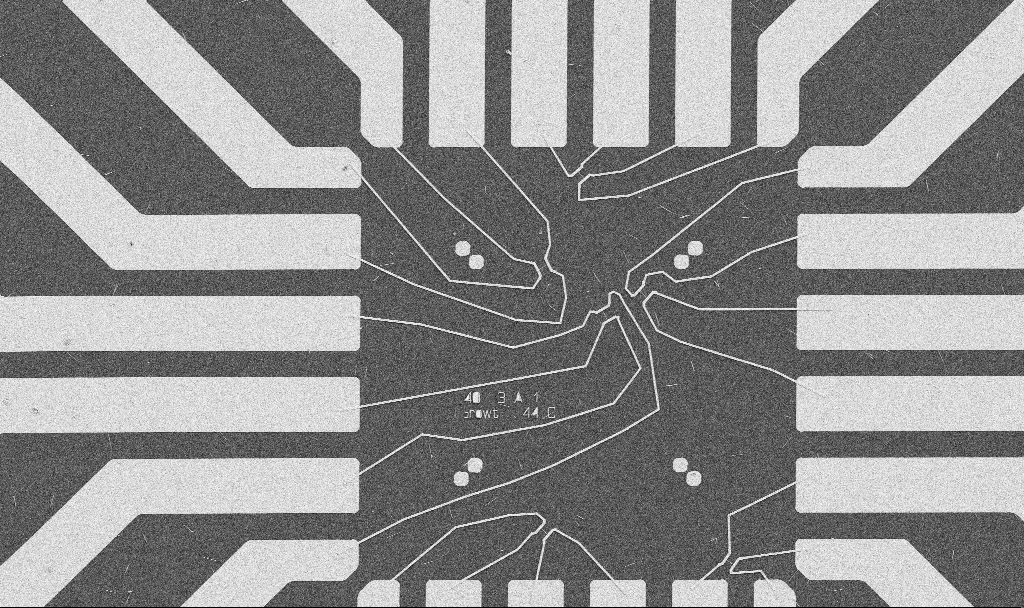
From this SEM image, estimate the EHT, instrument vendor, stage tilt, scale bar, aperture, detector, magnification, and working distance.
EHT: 5 kV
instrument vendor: Zeiss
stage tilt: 0°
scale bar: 20000 nm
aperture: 30 µm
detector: SE2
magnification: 1 K X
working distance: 10.7 mm